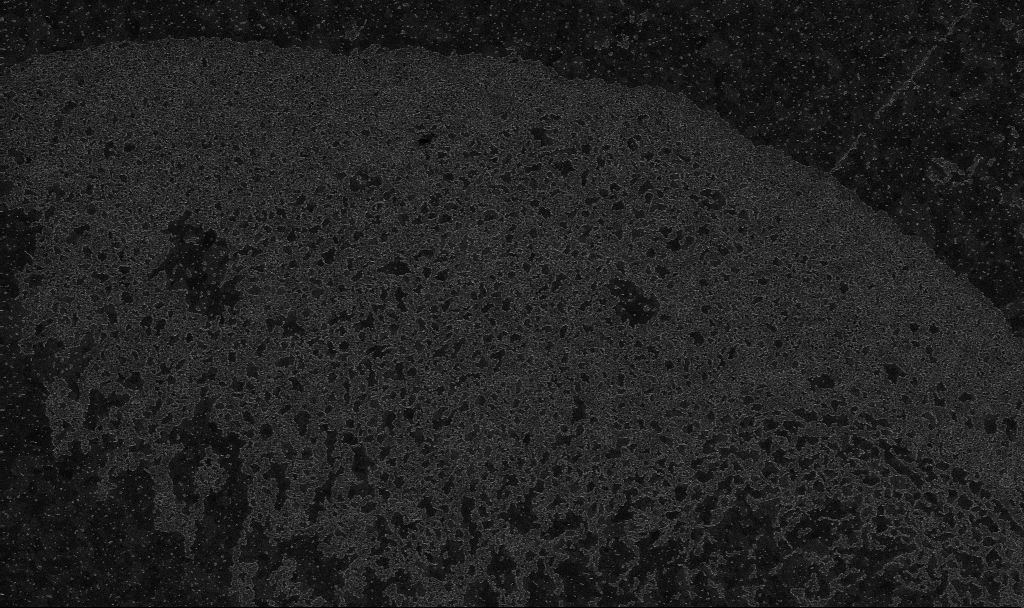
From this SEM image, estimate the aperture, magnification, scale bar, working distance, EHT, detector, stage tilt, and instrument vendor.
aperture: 30 µm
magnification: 20 K X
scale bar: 1000 nm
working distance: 3.4 mm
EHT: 10 kV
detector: InLens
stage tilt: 0°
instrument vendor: Zeiss